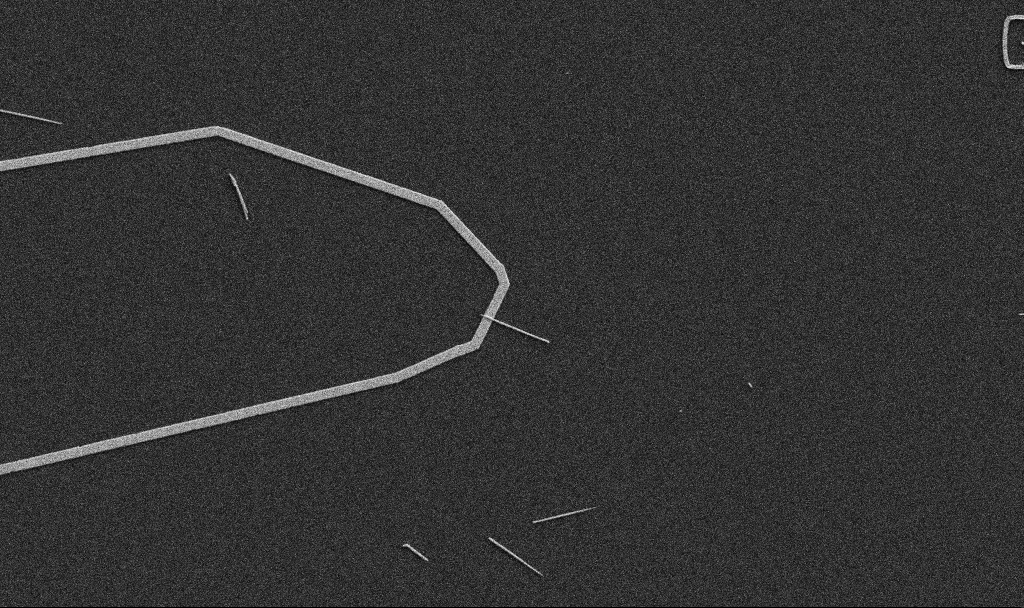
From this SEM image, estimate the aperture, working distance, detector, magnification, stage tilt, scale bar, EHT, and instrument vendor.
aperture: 30 µm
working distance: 10.7 mm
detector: SE2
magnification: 5 K X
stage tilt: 0°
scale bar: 10000 nm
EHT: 5 kV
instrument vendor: Zeiss